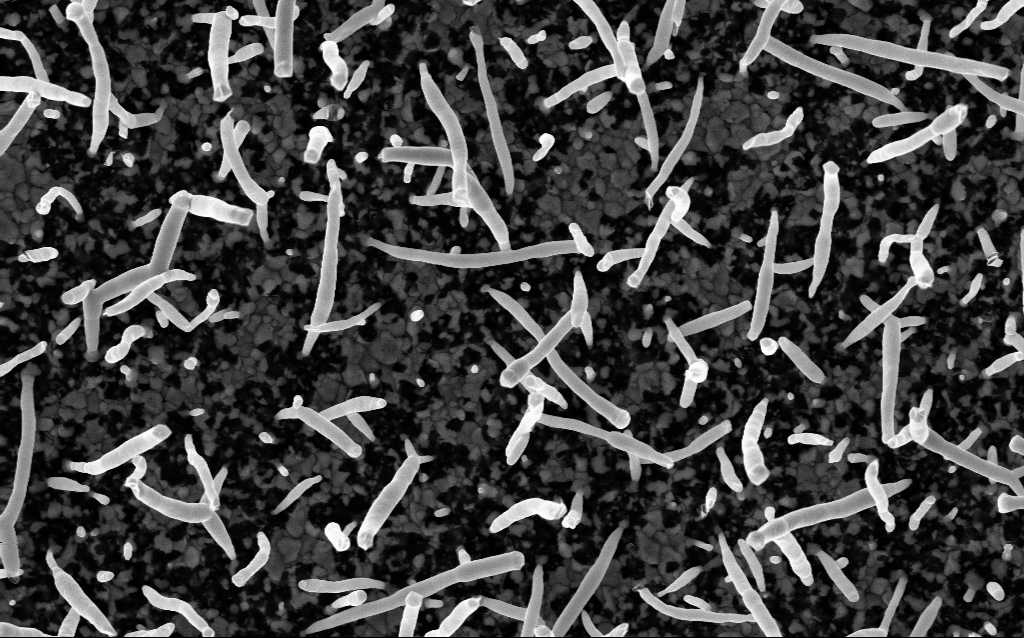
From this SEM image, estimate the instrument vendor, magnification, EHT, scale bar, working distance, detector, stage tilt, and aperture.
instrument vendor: Zeiss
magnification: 50 K X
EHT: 5 kV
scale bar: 1000 nm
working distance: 2.6 mm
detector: InLens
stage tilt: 0°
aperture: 30 µm